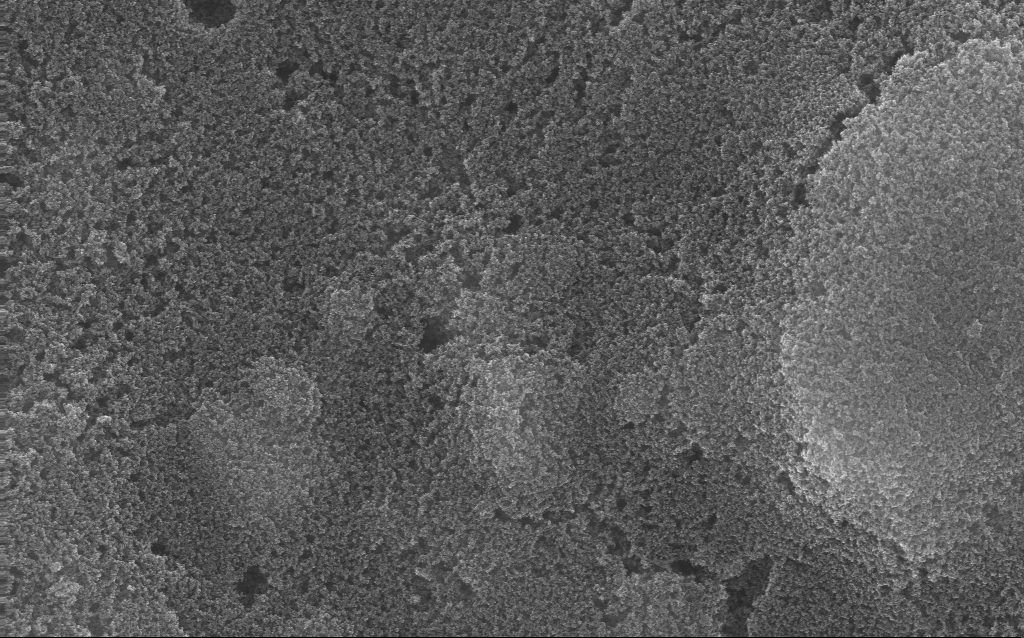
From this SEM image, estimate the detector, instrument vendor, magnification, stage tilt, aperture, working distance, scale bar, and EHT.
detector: InLens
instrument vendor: Zeiss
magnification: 23.9 K X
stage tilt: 0°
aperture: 30 µm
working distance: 3 mm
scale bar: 1000 nm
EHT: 20 kV